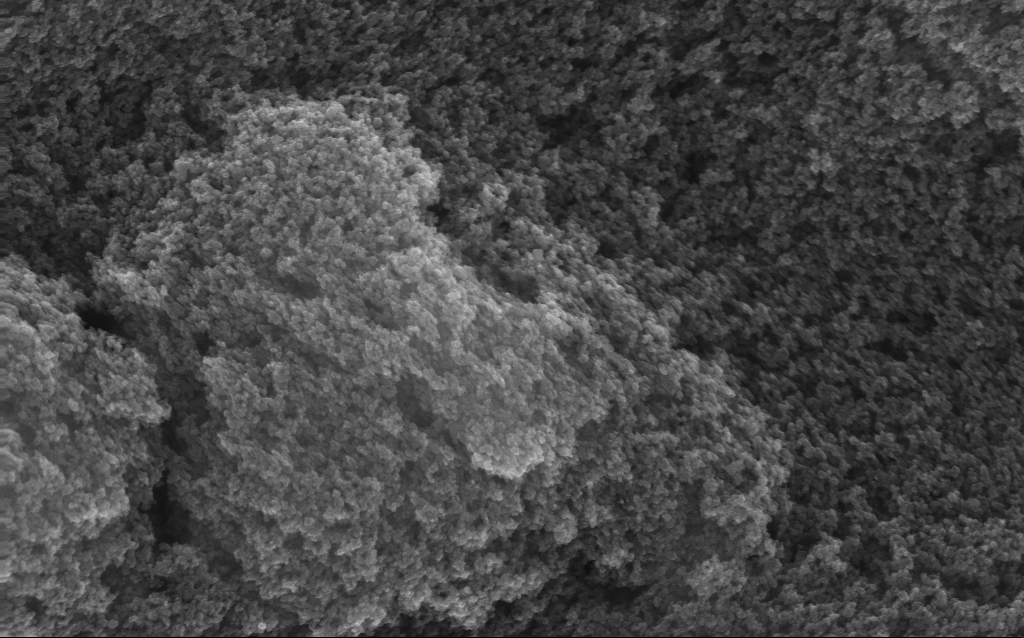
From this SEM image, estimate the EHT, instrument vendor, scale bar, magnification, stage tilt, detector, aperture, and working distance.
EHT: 15 kV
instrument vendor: Zeiss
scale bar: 1000 nm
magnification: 65.04 K X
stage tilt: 0°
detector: InLens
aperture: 20 µm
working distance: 2.7 mm